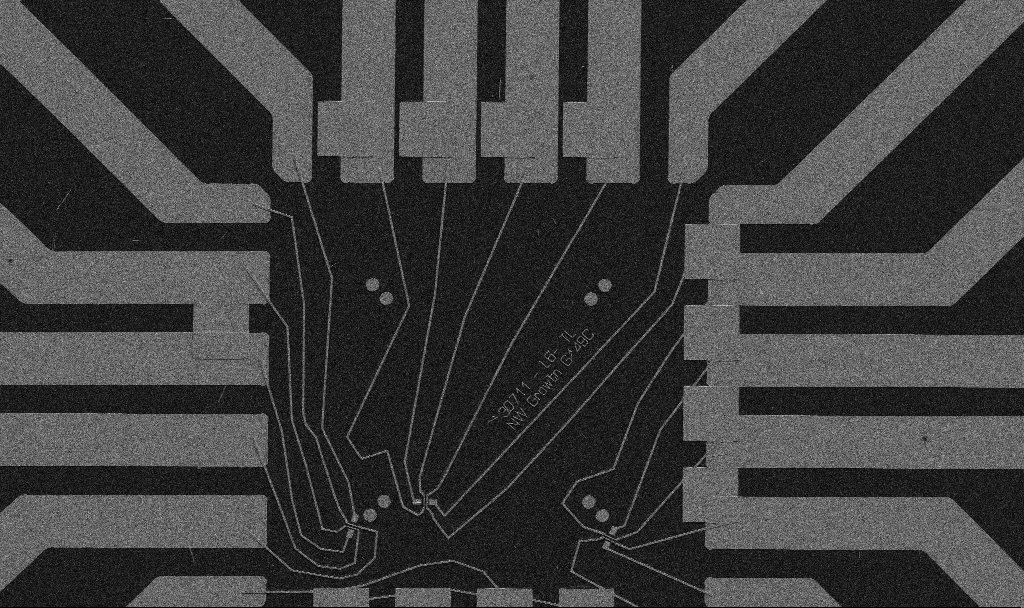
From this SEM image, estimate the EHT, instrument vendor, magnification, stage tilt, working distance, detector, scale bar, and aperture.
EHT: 5 kV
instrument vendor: Zeiss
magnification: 1 K X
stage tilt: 0°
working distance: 10.7 mm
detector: SE2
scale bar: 20000 nm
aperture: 30 µm